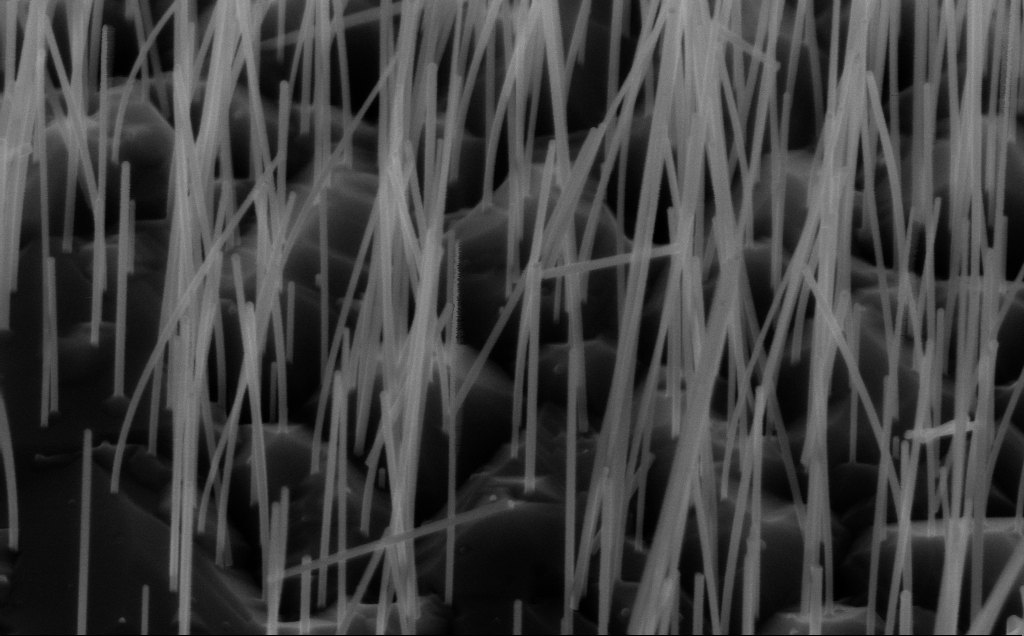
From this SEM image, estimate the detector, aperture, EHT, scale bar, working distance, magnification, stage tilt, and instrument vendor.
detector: InLens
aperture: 30 µm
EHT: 10 kV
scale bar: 200 nm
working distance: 5 mm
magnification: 80 K X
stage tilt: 45°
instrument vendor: Zeiss